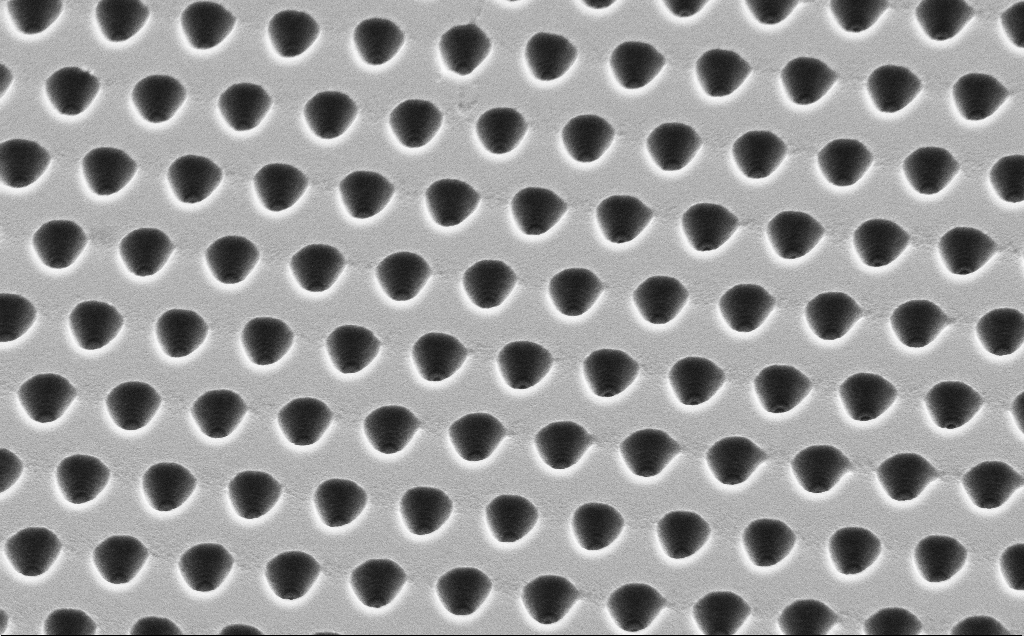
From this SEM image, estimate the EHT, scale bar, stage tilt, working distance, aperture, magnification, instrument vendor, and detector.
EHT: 5 kV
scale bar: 2000 nm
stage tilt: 45°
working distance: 10 mm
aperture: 30 µm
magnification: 7.85 K X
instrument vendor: Zeiss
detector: SE2